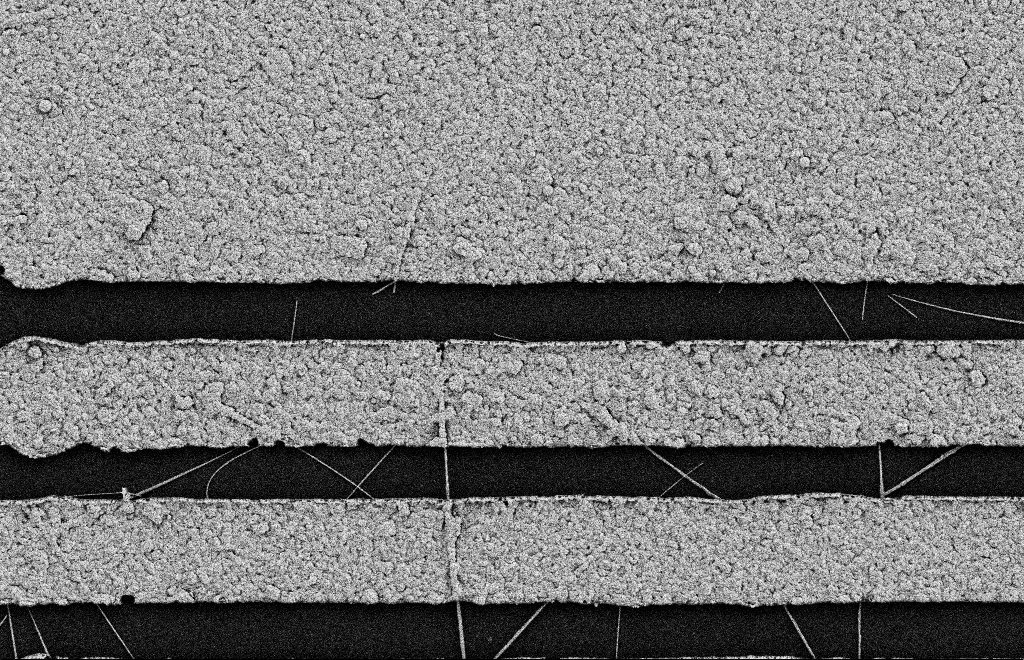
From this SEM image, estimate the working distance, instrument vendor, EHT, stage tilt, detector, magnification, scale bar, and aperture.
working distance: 11 mm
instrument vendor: Zeiss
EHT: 2 kV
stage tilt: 0°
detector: SE2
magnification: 14.47 K X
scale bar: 1000 nm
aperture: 20 µm